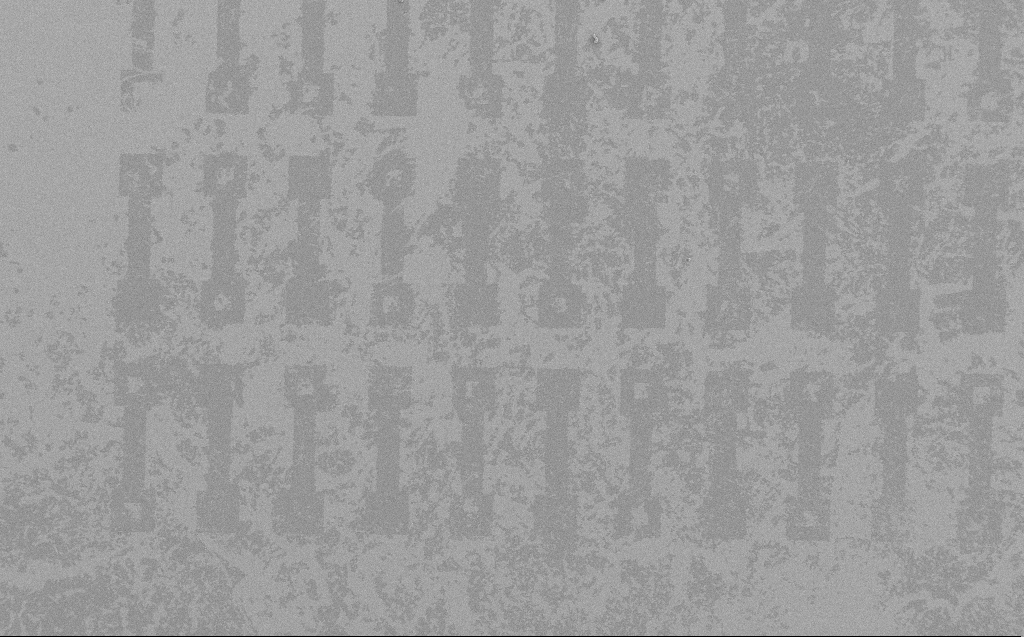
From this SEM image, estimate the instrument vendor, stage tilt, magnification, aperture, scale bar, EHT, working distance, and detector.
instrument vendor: Zeiss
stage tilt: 0°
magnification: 0.313 K X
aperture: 30 µm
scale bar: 100000 nm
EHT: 3 kV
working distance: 5 mm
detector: SE2